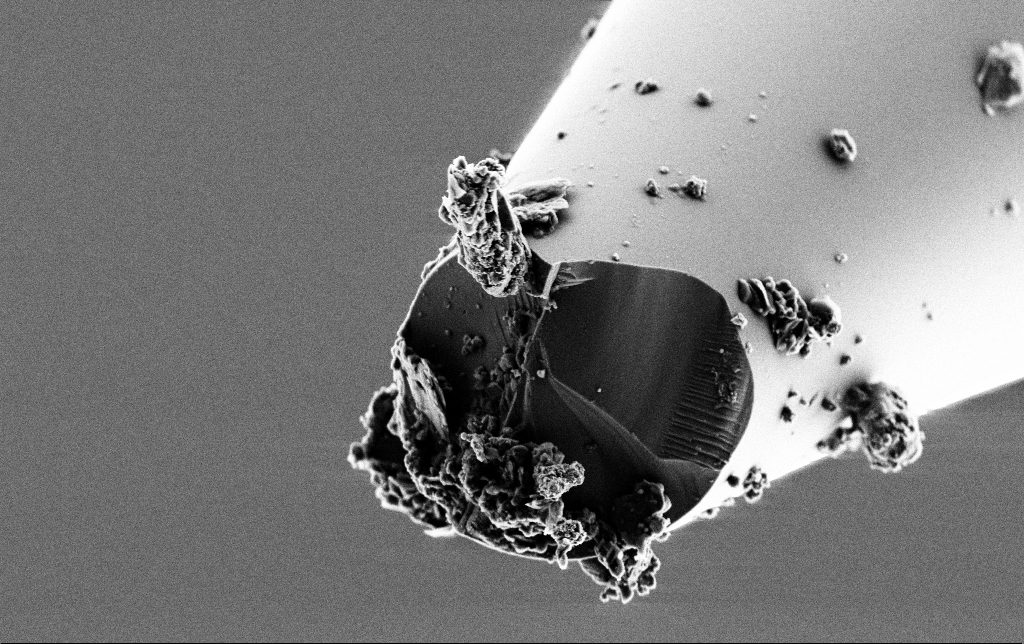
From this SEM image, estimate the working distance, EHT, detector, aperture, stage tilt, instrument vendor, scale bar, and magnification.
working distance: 6.2 mm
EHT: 2 kV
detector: SE2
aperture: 30 µm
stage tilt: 70°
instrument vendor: Zeiss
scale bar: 2000 nm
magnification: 15.82 K X